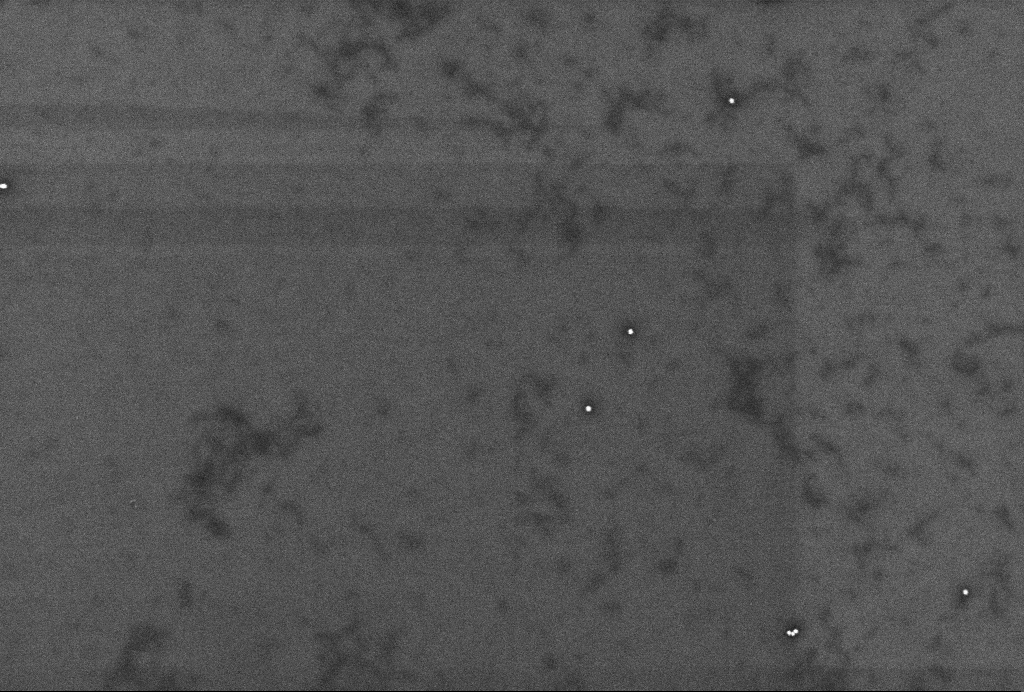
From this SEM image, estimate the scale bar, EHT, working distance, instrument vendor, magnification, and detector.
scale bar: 200 nm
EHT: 2 kV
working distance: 3.3 mm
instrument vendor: Zeiss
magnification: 64.42 K X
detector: InLens